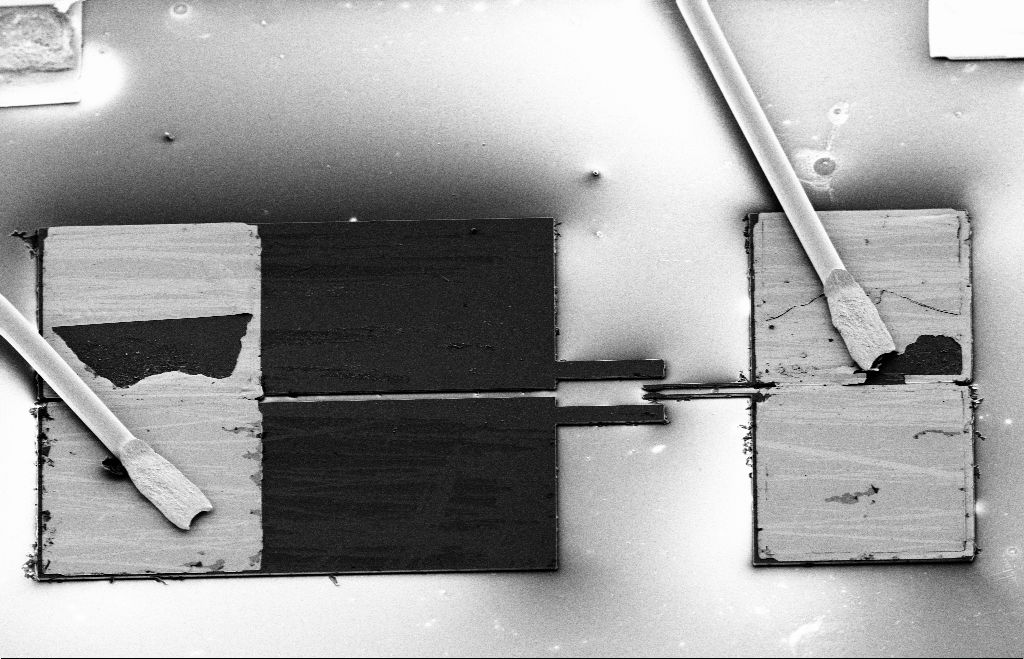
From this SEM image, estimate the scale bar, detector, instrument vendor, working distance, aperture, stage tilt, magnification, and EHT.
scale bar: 100000 nm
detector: SE2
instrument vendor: Zeiss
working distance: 13 mm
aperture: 30 µm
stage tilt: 40°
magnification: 0.399 K X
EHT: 5 kV